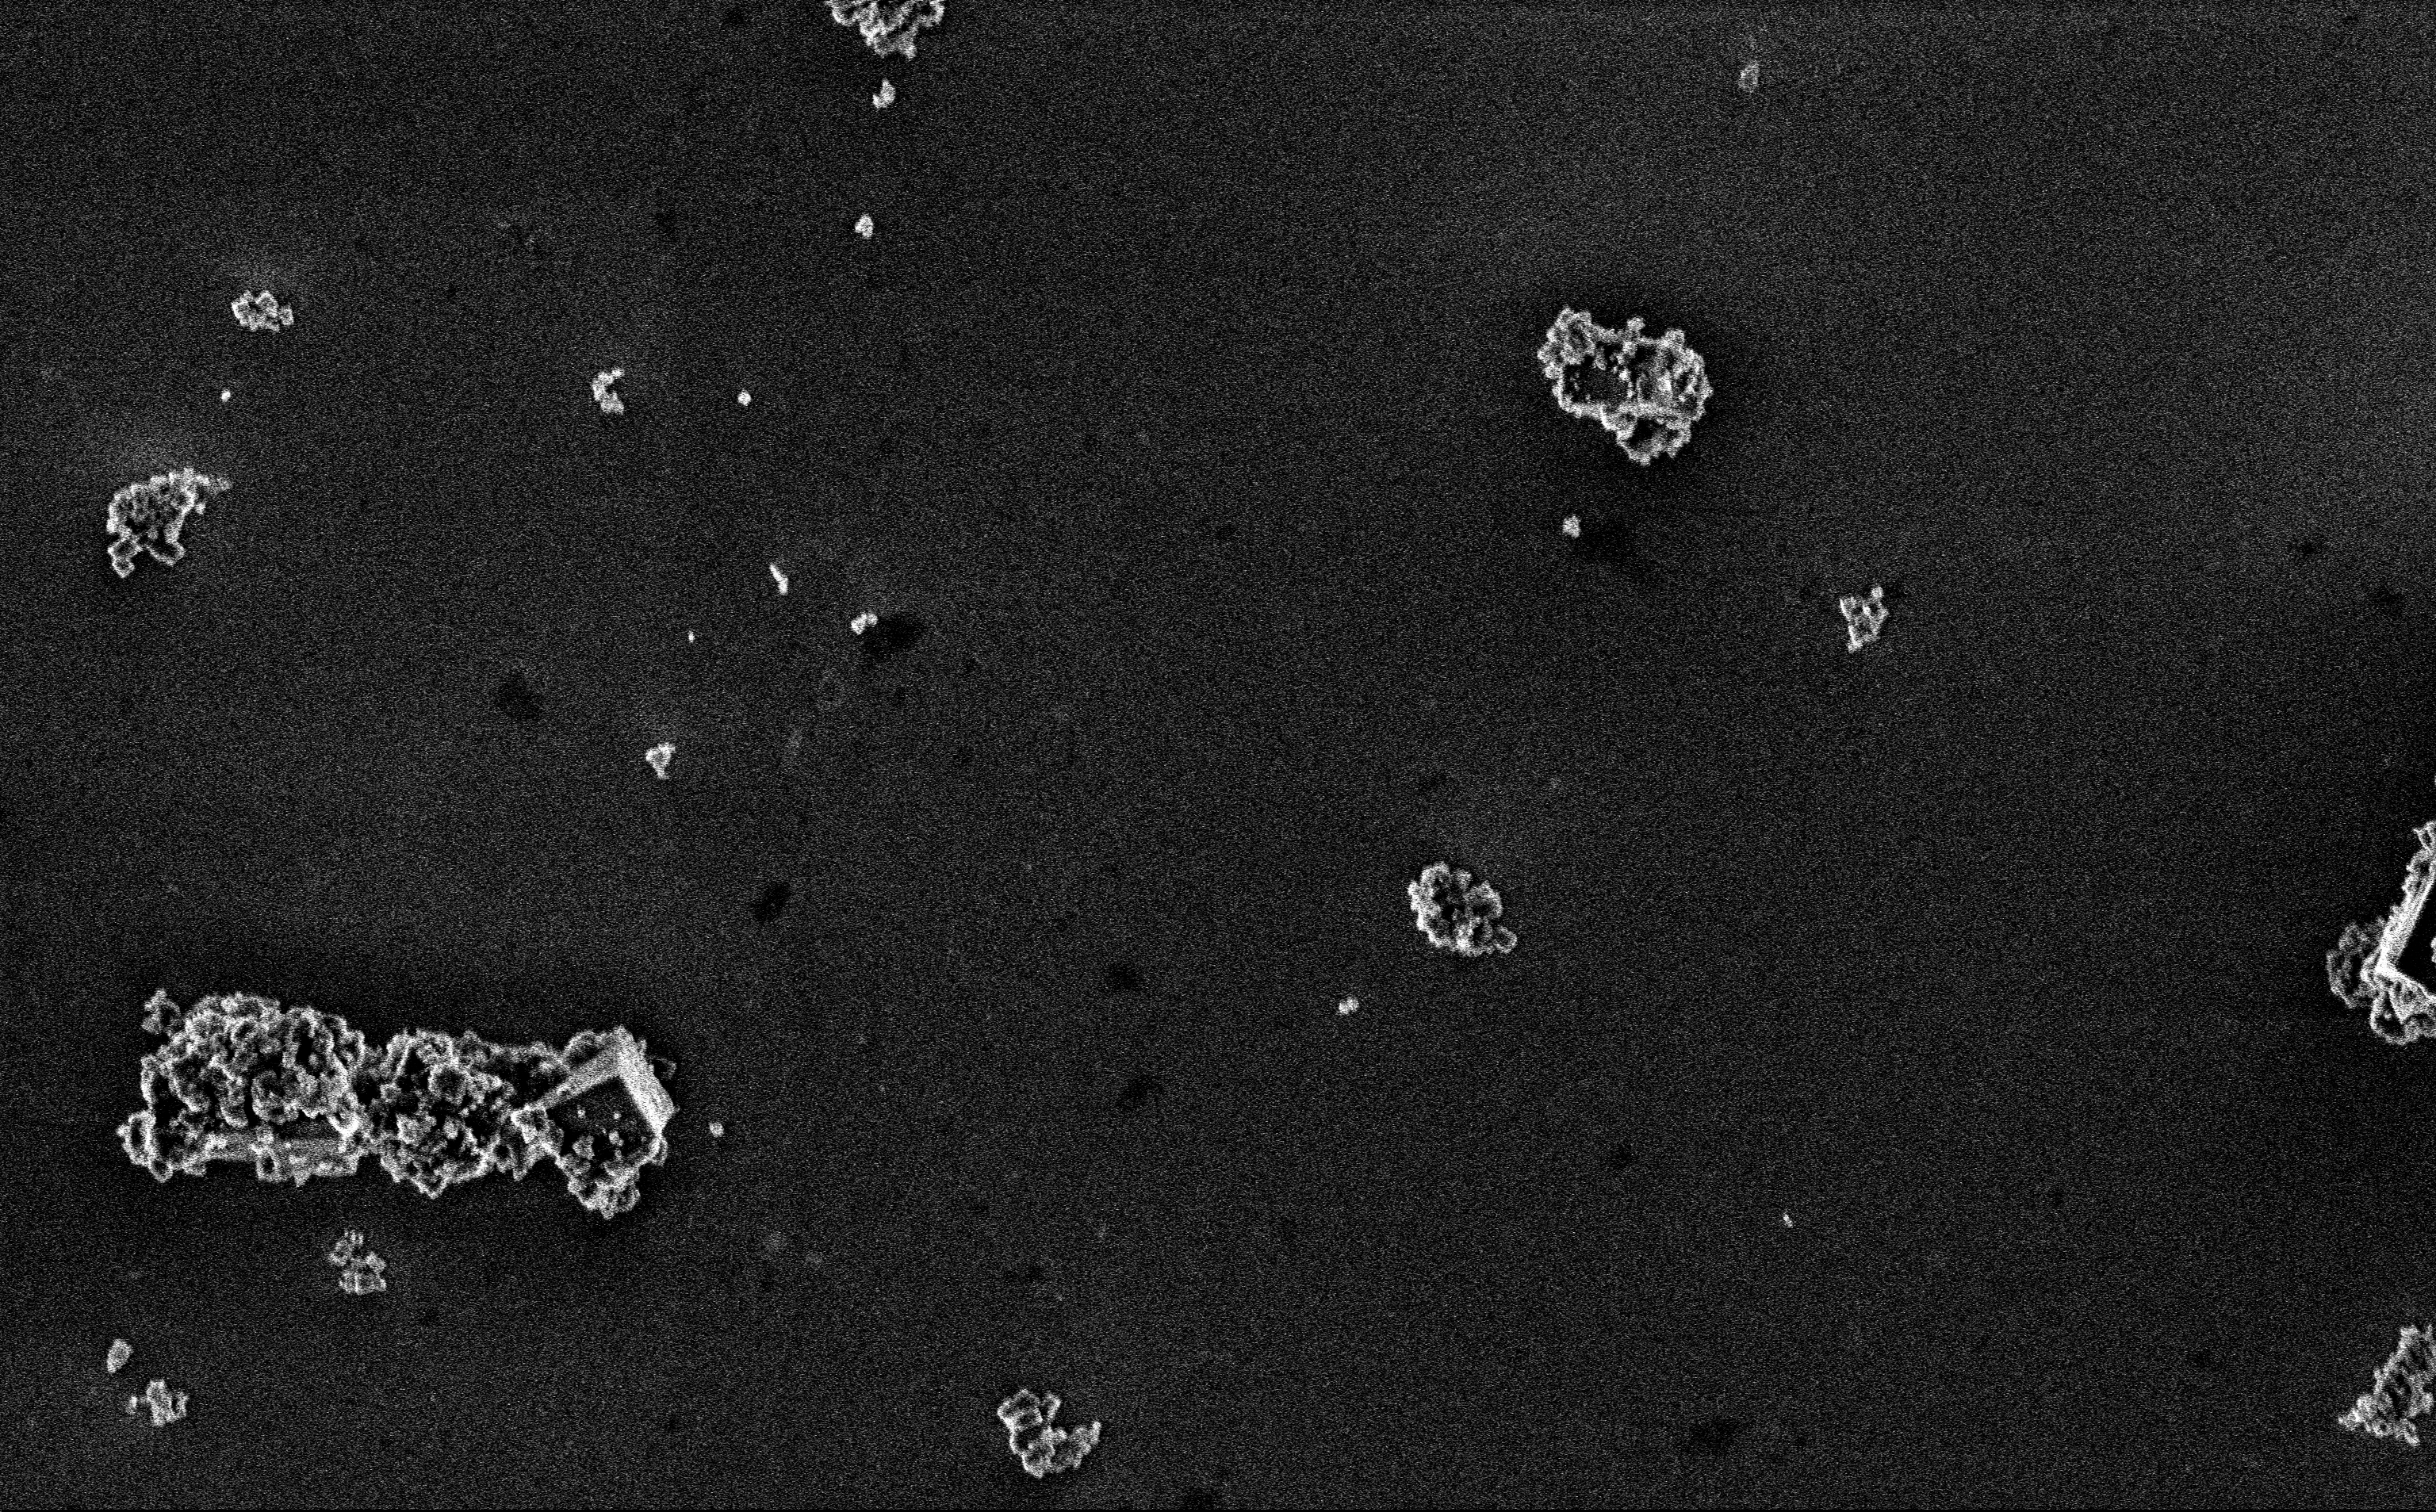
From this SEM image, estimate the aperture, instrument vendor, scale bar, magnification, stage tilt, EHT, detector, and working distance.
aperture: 30 µm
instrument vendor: Zeiss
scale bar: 2000 nm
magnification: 11.15 K X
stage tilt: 0°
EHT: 3 kV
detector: InLens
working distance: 3 mm